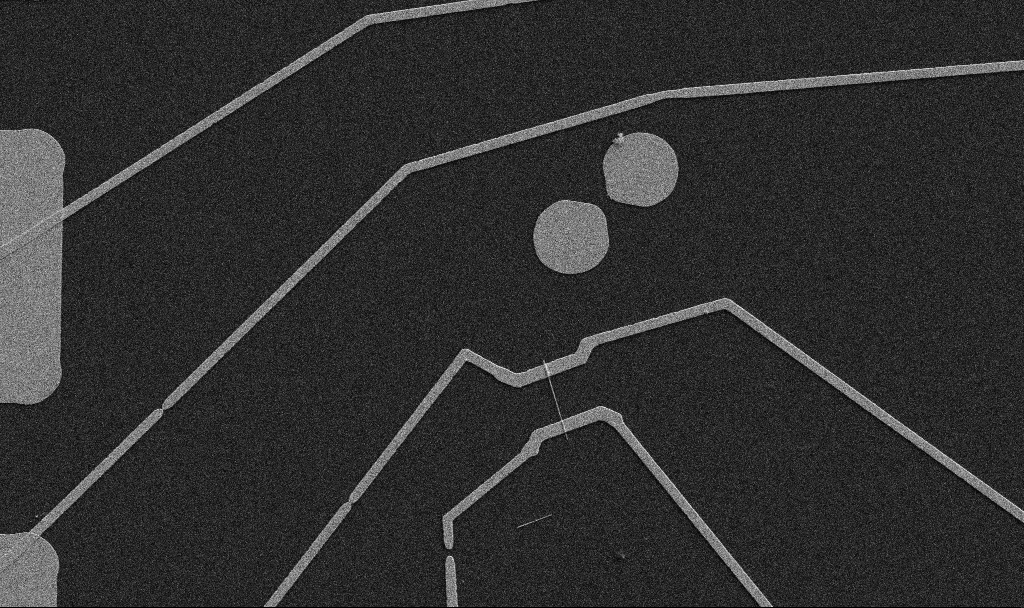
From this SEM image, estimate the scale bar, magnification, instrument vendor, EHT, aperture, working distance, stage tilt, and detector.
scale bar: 10000 nm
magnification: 5 K X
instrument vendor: Zeiss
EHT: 5 kV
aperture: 30 µm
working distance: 10.7 mm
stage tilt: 0°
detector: SE2